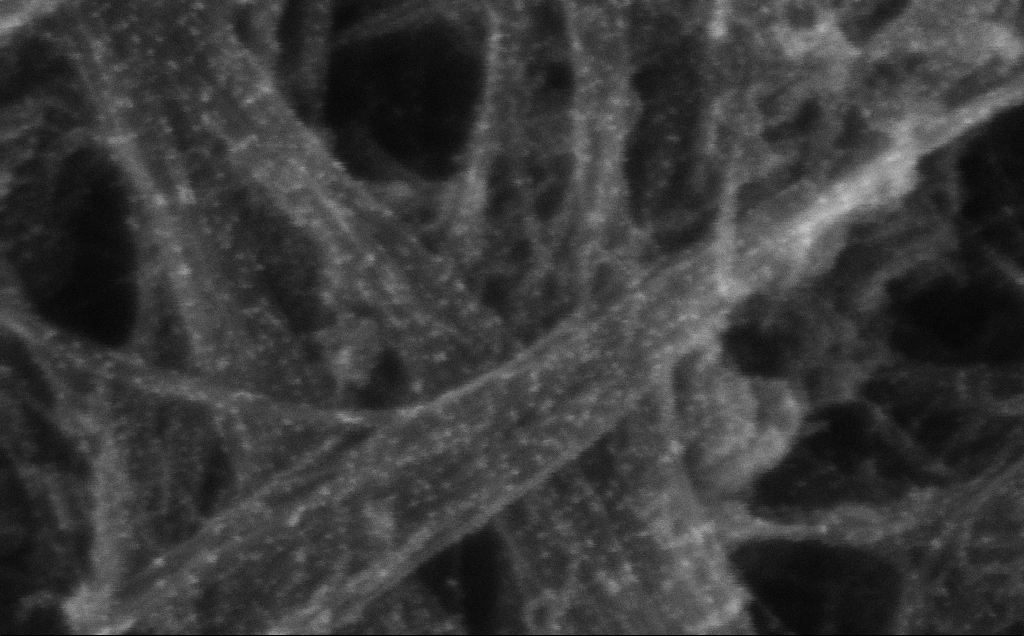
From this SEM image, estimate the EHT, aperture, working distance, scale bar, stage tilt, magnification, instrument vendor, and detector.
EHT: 10 kV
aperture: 30 µm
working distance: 3 mm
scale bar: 100 nm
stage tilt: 0°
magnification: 484.67 K X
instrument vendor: Zeiss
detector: InLens